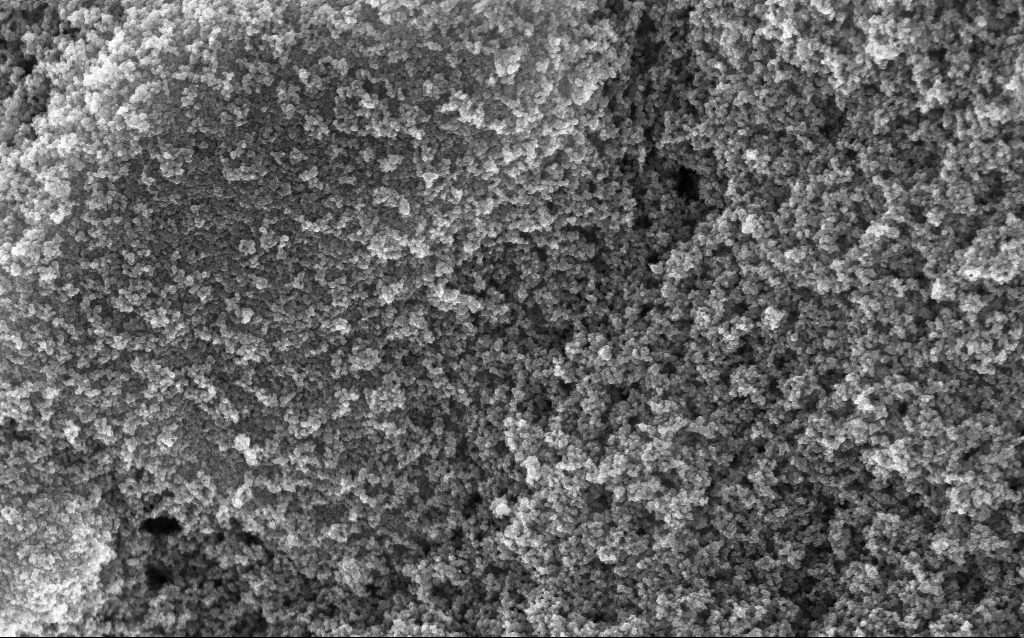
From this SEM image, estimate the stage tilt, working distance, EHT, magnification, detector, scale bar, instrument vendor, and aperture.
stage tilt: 0°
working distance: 2.6 mm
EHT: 10 kV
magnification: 65.04 K X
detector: InLens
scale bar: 1000 nm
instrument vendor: Zeiss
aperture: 30 µm